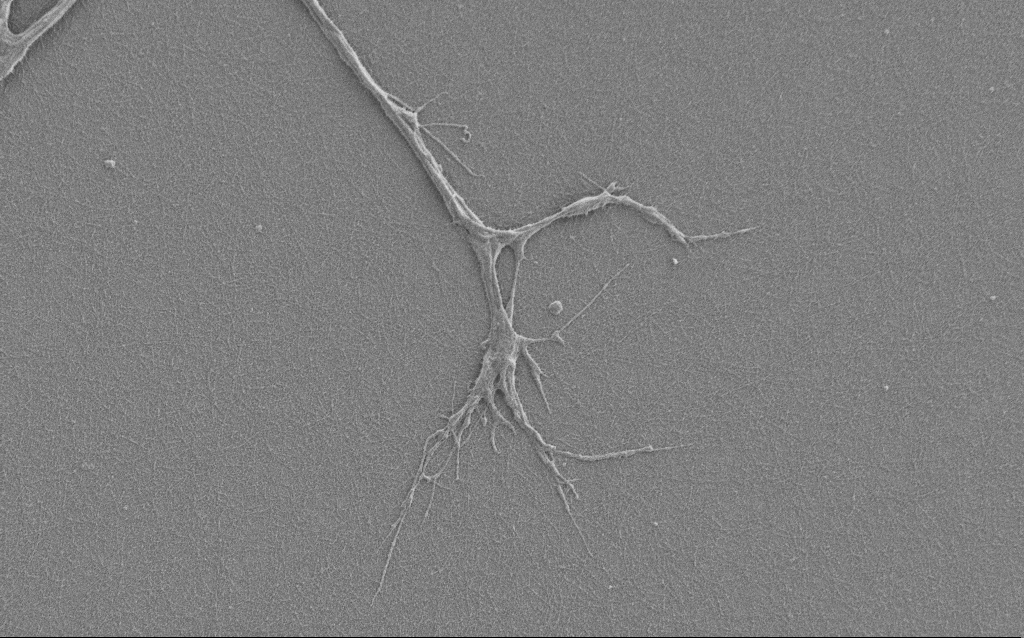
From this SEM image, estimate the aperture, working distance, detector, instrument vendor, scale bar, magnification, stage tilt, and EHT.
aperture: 30 µm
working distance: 6 mm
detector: SE2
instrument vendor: Zeiss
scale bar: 10000 nm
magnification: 6 K X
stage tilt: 0°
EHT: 1 kV